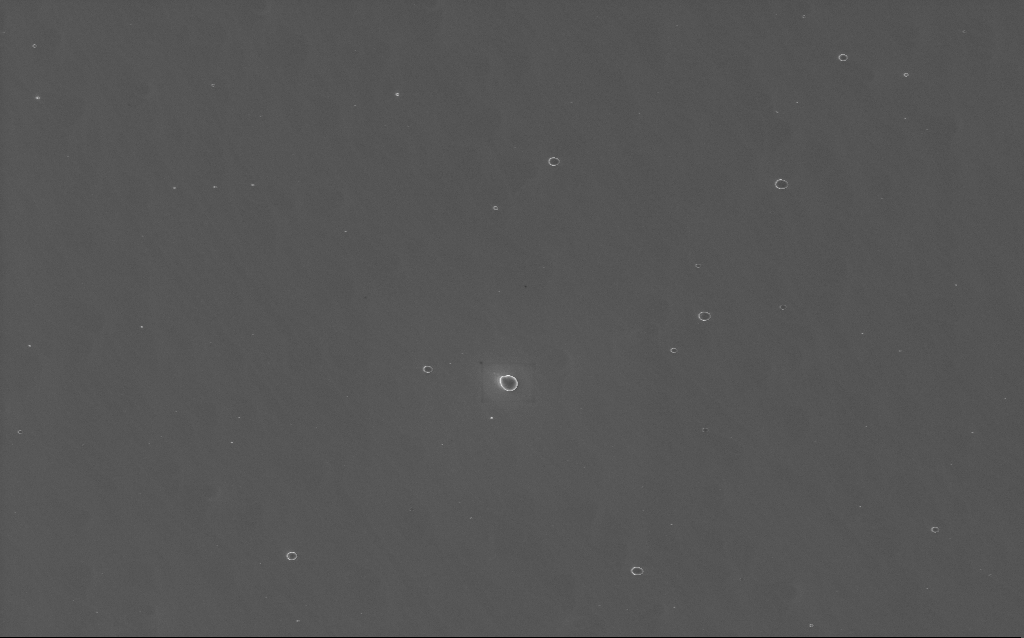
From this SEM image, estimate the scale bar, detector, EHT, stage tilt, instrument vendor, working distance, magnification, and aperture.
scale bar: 10000 nm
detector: InLens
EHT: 10 kV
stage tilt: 0°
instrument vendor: Zeiss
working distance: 2 mm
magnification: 3.81 K X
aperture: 30 µm